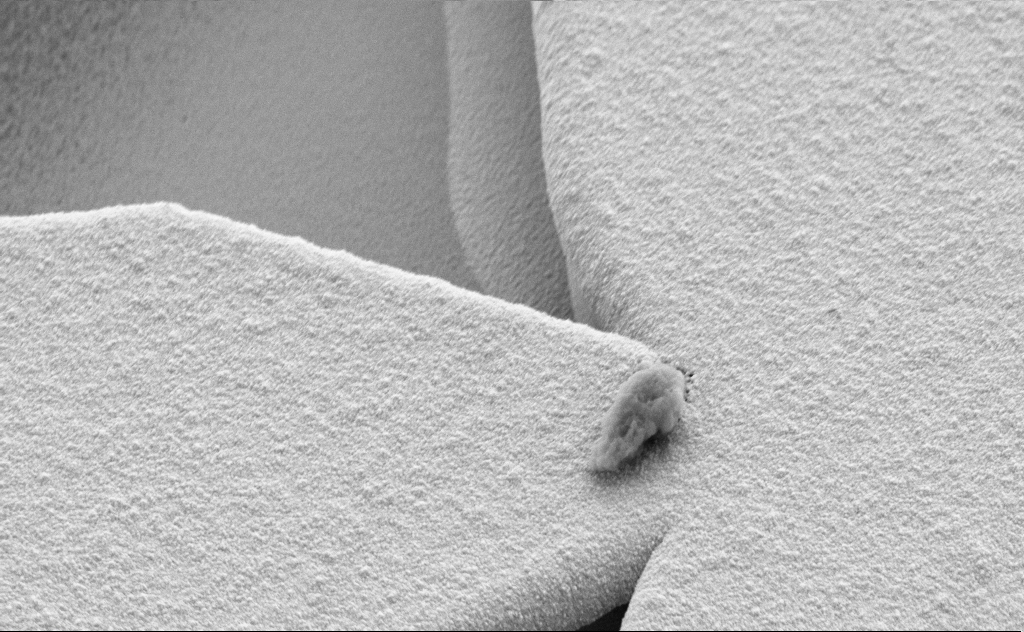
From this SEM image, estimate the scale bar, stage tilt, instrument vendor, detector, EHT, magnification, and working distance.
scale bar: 2000 nm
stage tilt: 45°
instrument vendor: Zeiss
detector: SE2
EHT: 5 kV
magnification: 19.62 K X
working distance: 8 mm